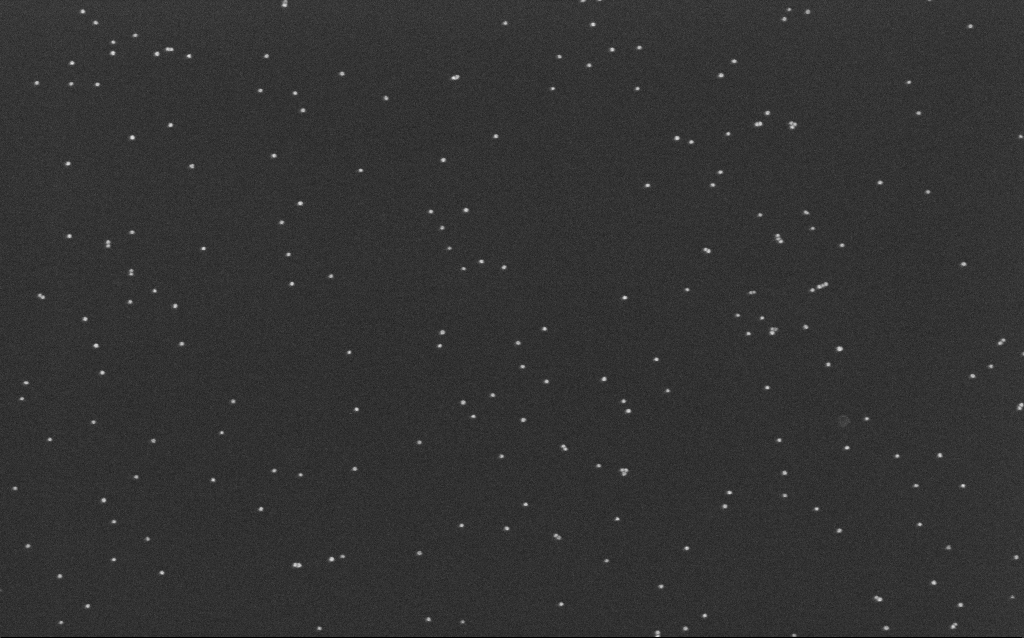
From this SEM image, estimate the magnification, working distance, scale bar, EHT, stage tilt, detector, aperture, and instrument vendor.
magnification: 100 K X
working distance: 6.6 mm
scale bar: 200 nm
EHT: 10 kV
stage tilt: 0°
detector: InLens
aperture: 30 µm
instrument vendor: Zeiss